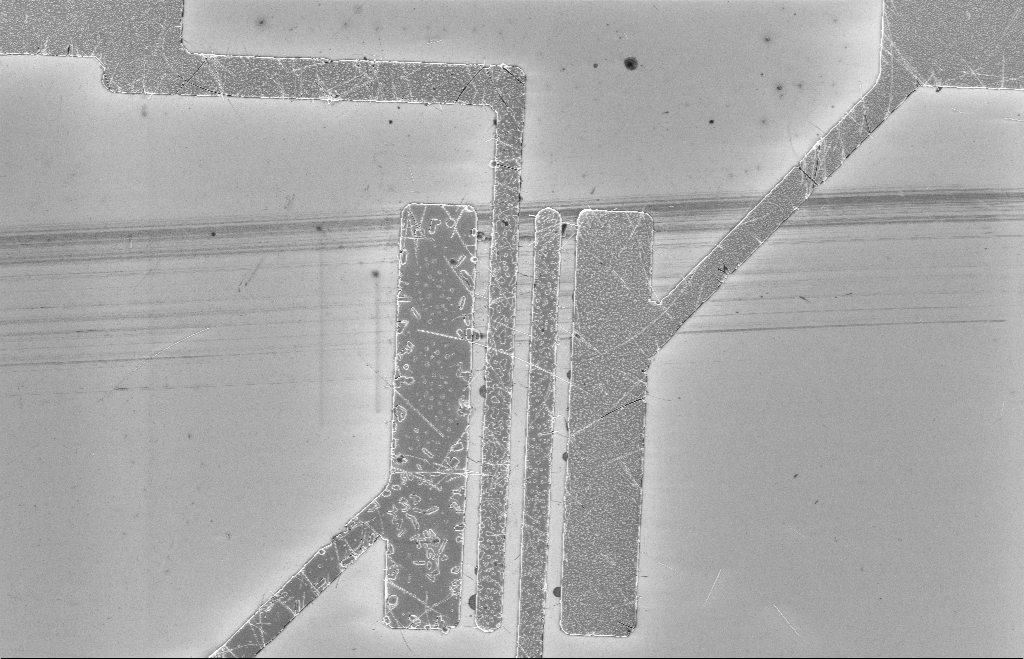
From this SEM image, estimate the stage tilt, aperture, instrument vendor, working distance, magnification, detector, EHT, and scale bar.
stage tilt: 0°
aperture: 20 µm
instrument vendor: Zeiss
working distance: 8 mm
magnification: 2.6 K X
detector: InLens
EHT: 5 kV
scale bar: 10000 nm